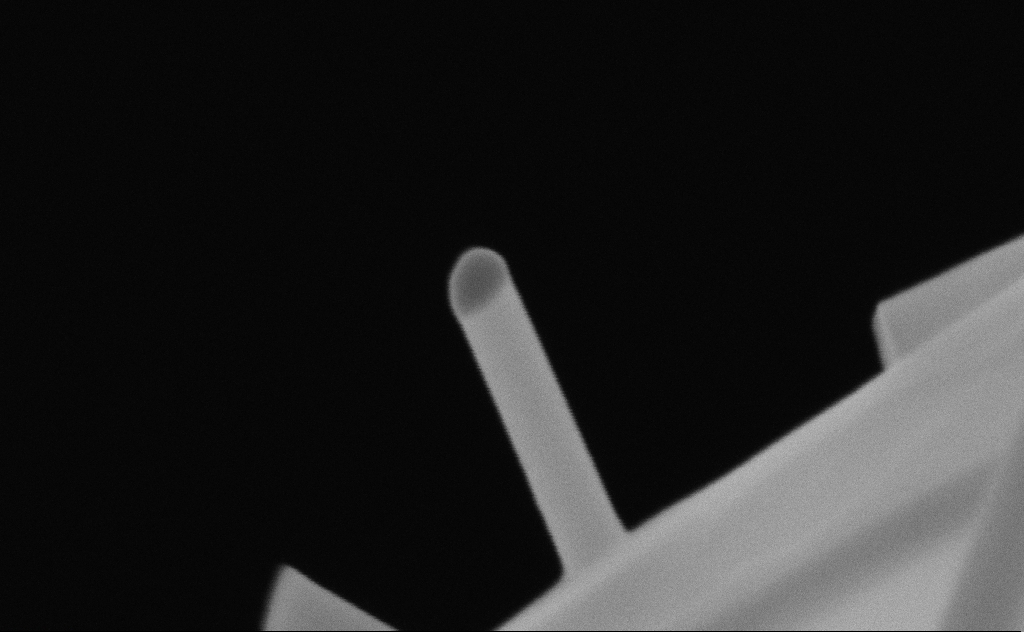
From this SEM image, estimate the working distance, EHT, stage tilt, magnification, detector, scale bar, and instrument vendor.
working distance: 9 mm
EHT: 20 kV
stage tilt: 0°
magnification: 325.29 K X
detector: SE2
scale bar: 200 nm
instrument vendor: Zeiss